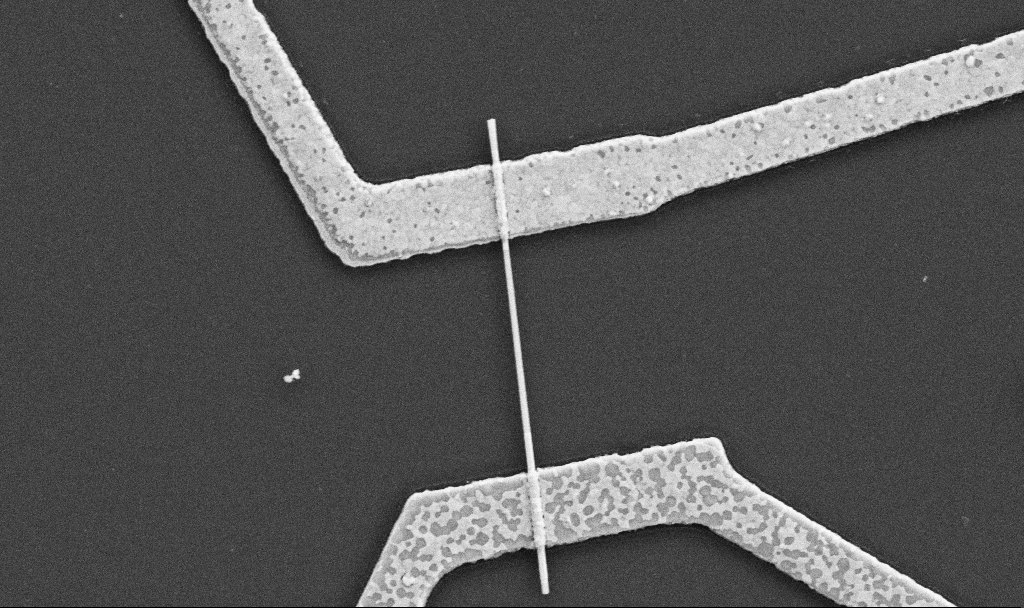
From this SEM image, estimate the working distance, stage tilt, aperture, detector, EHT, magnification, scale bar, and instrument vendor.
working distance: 8.7 mm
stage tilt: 0°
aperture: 30 µm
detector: SE2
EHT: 5 kV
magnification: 30 K X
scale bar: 1000 nm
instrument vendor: Zeiss